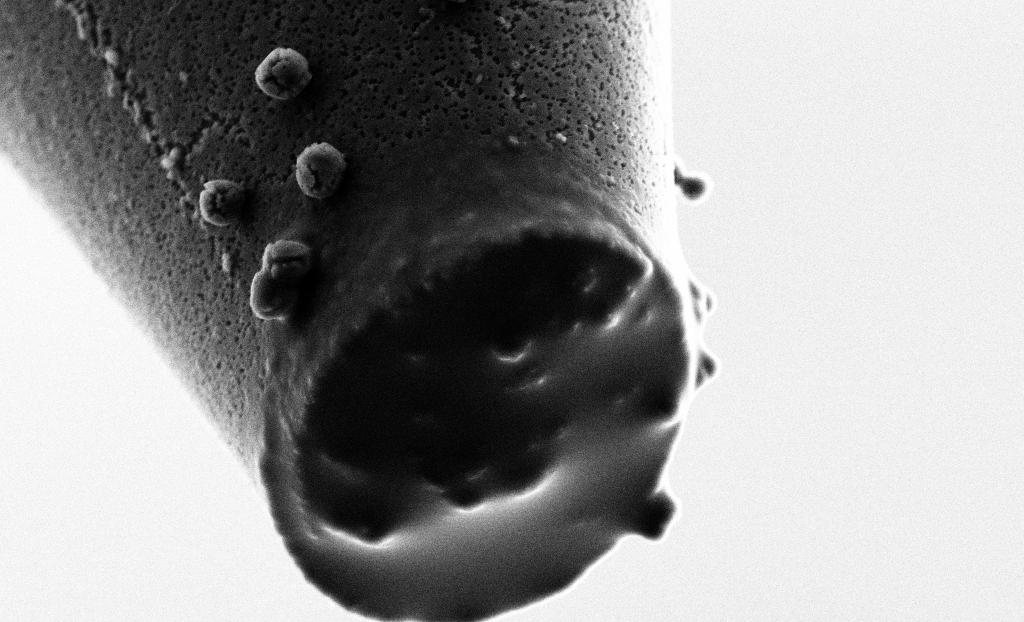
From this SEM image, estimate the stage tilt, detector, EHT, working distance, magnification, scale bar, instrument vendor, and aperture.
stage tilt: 43.9°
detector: SE2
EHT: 5 kV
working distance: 5.7 mm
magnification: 15 K X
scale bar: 1000 nm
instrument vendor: Zeiss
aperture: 30 µm